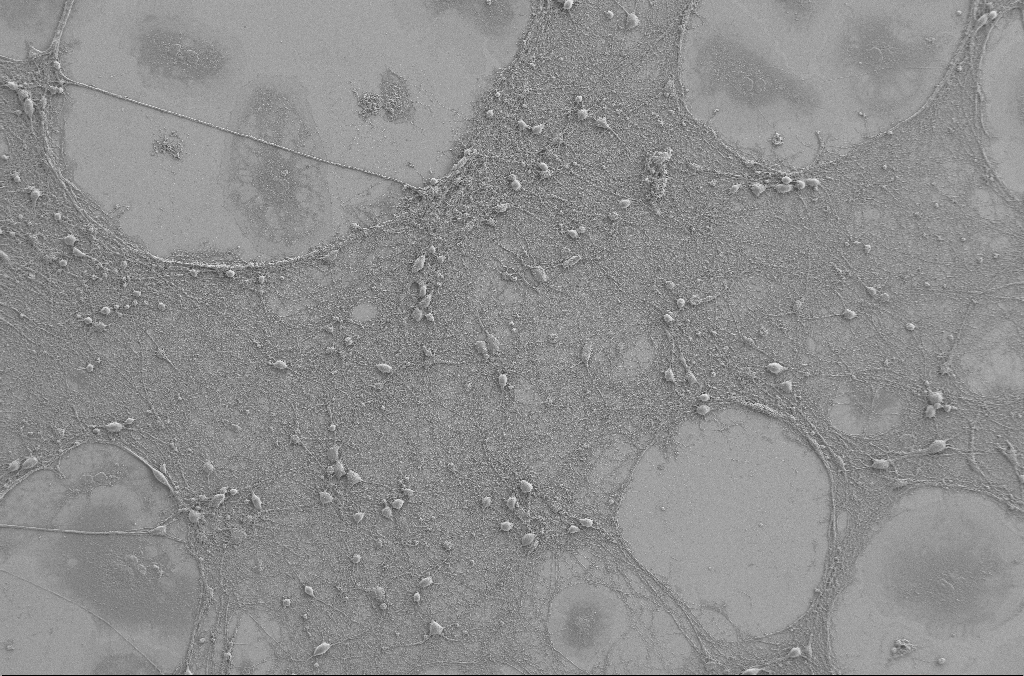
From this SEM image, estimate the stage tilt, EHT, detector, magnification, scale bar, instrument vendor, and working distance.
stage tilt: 0°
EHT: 2 kV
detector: SE2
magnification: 0.5 K X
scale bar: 100000 nm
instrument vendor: Zeiss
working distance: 4 mm